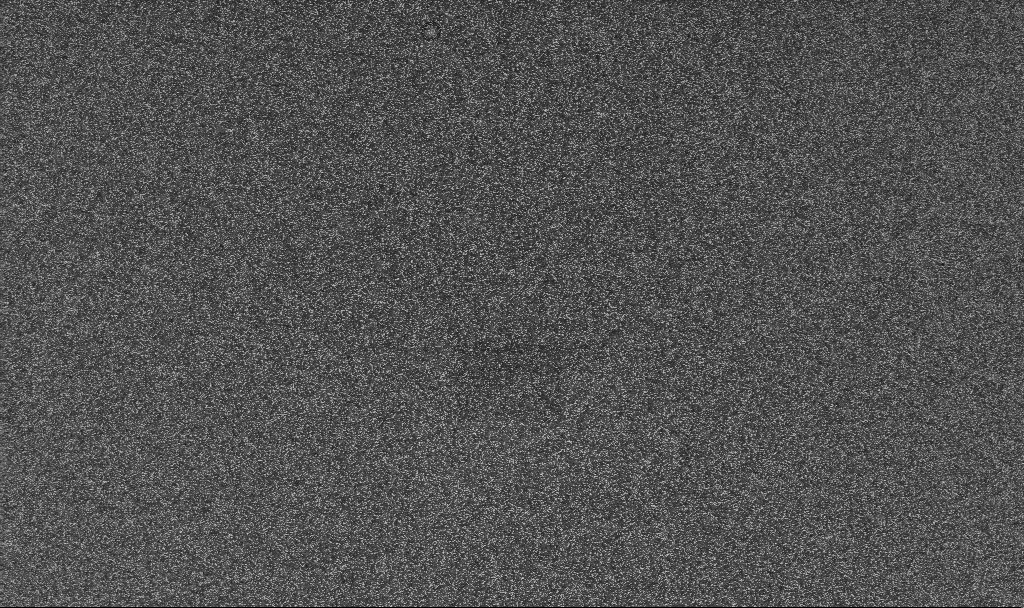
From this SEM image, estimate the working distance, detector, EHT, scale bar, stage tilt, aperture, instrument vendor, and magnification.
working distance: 3.3 mm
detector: InLens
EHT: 10 kV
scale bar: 10000 nm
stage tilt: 0°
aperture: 30 µm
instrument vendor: Zeiss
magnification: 5 K X